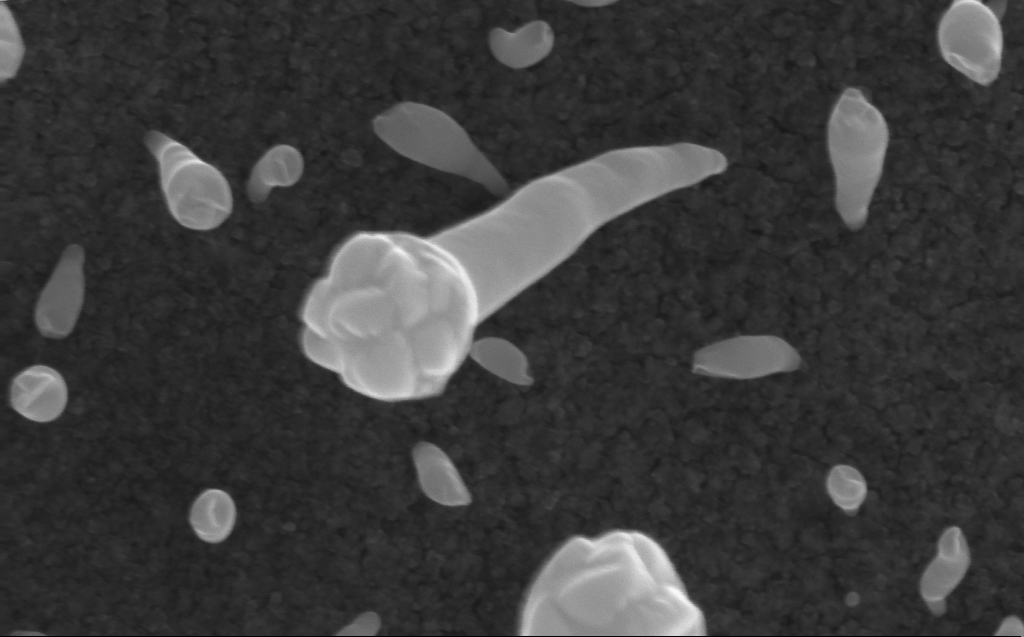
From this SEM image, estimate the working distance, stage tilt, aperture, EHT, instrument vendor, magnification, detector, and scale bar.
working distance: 3 mm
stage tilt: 0°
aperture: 30 µm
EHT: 10 kV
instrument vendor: Zeiss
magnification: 200 K X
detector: InLens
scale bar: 100 nm